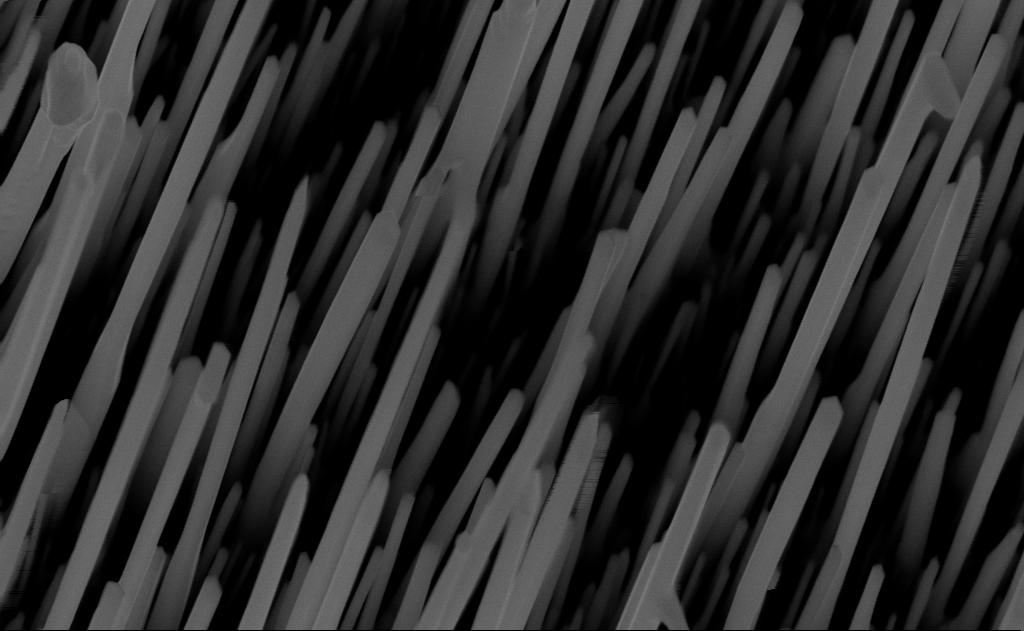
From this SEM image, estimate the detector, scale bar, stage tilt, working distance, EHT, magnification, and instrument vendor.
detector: InLens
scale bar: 200 nm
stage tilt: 0°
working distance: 7 mm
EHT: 10 kV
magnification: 80 K X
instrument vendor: Zeiss